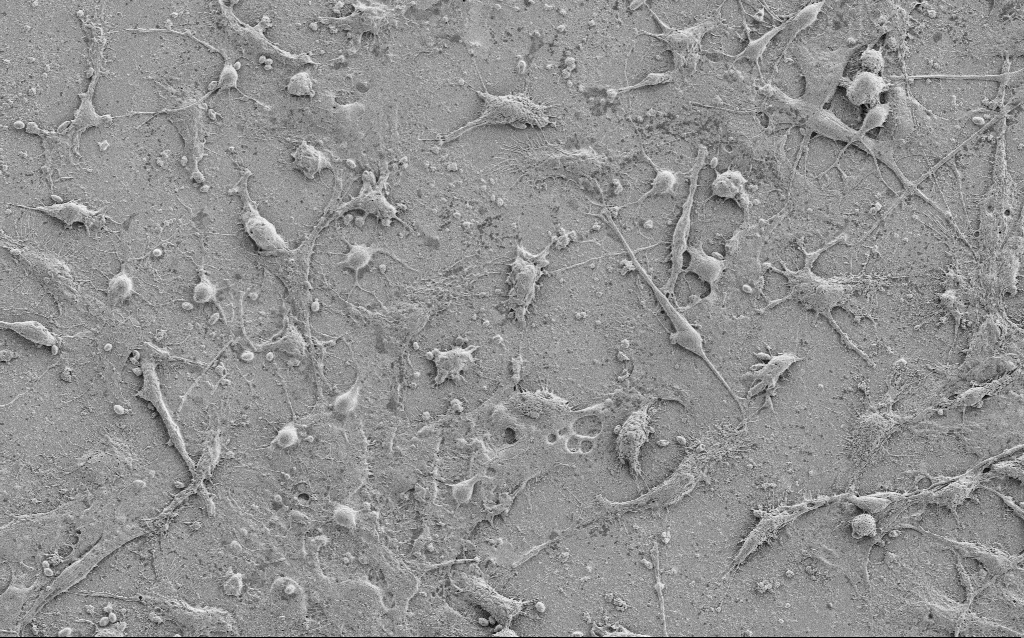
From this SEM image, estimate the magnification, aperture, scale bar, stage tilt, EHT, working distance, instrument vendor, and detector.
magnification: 1 K X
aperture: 30 µm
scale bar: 20000 nm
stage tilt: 0°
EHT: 2 kV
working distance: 6.9 mm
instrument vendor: Zeiss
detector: SE2